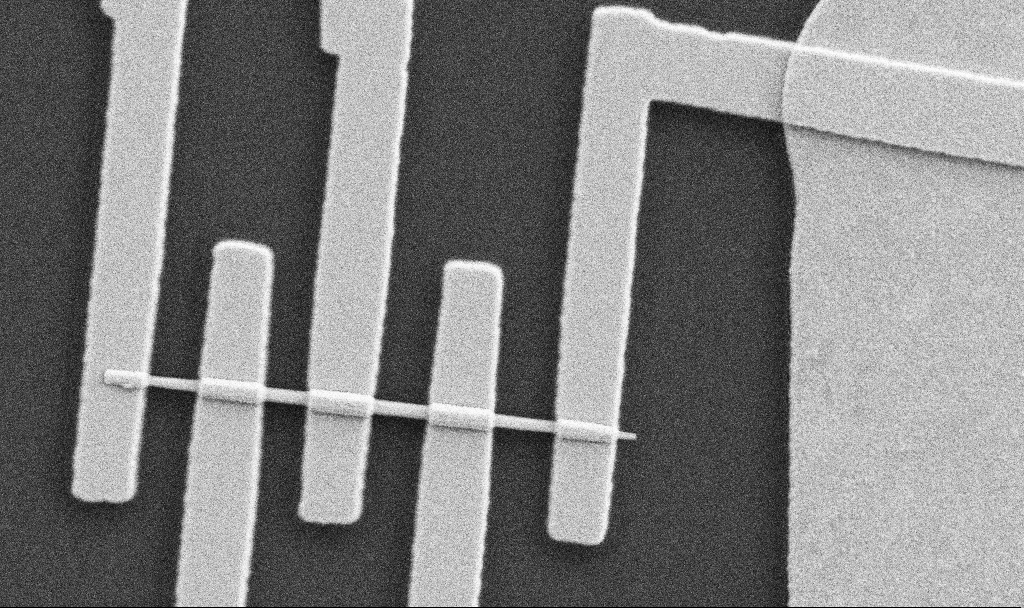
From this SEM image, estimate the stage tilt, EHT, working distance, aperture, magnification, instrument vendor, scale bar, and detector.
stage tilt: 0°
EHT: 5 kV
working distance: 10.7 mm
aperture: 30 µm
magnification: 38.45 K X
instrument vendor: Zeiss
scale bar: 1000 nm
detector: SE2